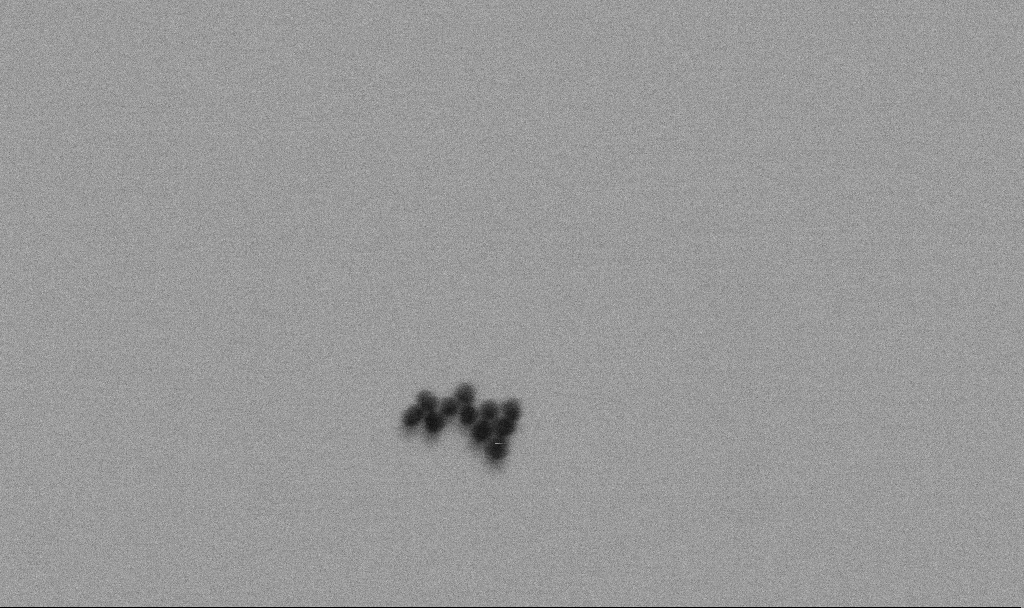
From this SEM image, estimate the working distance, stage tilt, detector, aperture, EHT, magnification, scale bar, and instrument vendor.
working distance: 4 mm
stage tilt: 0°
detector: SE2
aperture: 30 µm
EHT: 4 kV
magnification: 400 K X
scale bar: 100 nm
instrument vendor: Zeiss